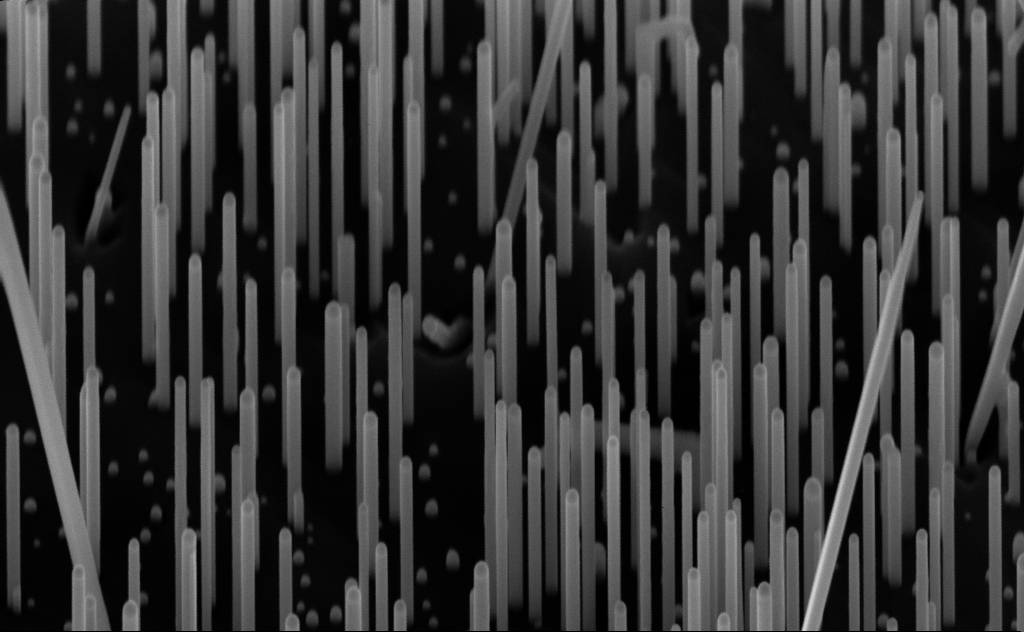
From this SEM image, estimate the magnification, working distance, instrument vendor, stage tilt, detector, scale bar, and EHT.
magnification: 80 K X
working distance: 7 mm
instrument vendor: Zeiss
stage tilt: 45°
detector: InLens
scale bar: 200 nm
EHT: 10 kV